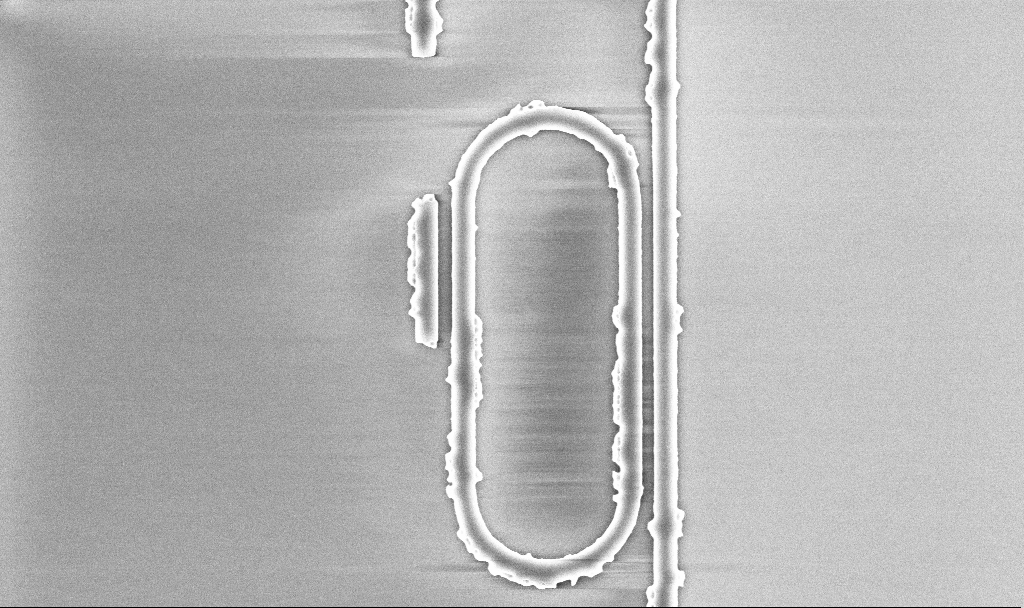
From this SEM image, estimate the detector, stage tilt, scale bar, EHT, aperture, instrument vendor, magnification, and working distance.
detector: InLens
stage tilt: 0°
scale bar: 2000 nm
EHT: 5 kV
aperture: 30 µm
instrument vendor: Zeiss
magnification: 17.8 K X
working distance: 5.2 mm